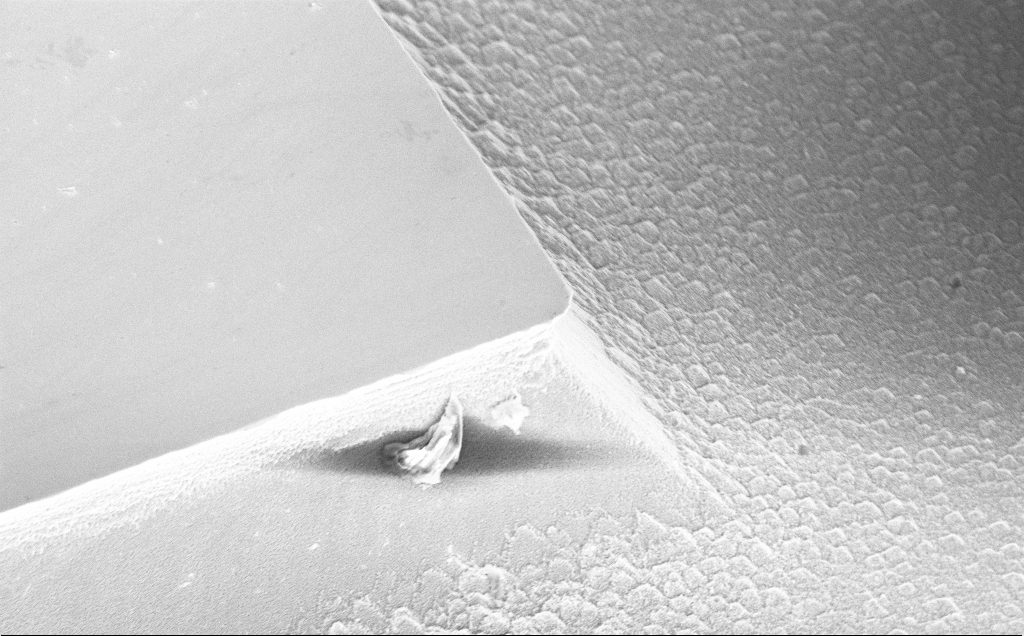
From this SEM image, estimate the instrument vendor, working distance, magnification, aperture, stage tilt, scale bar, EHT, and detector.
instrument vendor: Zeiss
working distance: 10 mm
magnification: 6.06 K X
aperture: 30 µm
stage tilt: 45°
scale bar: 10000 nm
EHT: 5 kV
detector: InLens